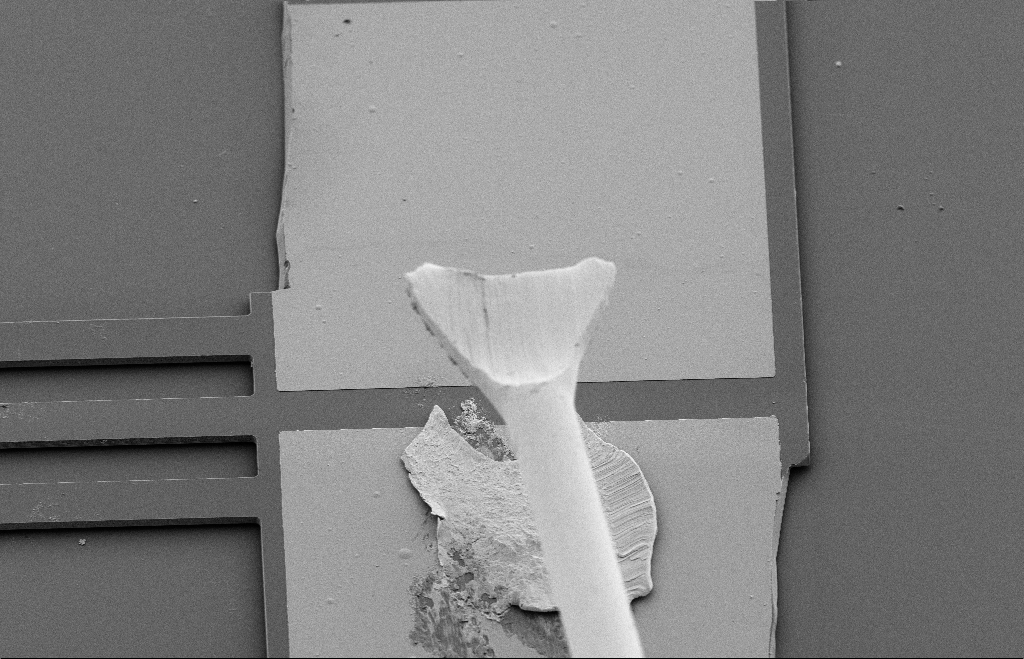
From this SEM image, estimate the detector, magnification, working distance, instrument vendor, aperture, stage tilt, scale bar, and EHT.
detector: SE2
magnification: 1.02 K X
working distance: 9 mm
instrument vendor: Zeiss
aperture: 20 µm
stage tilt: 39°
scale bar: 20000 nm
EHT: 5 kV